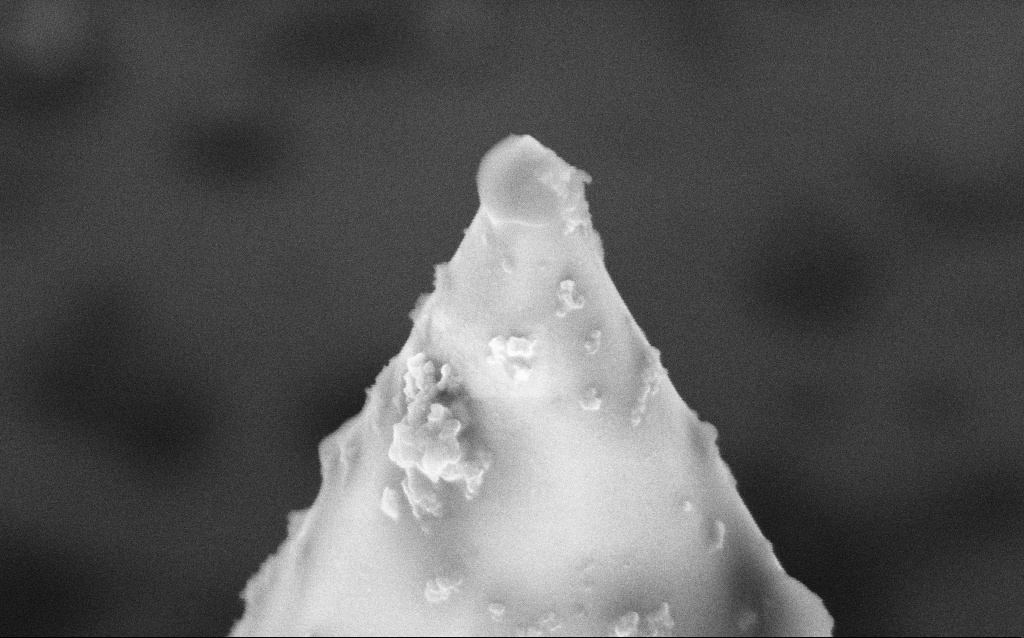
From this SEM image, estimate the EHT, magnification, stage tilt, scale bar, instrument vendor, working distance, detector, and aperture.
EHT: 5 kV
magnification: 86.35 K X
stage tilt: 45°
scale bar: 200 nm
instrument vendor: Zeiss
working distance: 11.8 mm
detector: InLens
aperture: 30 µm